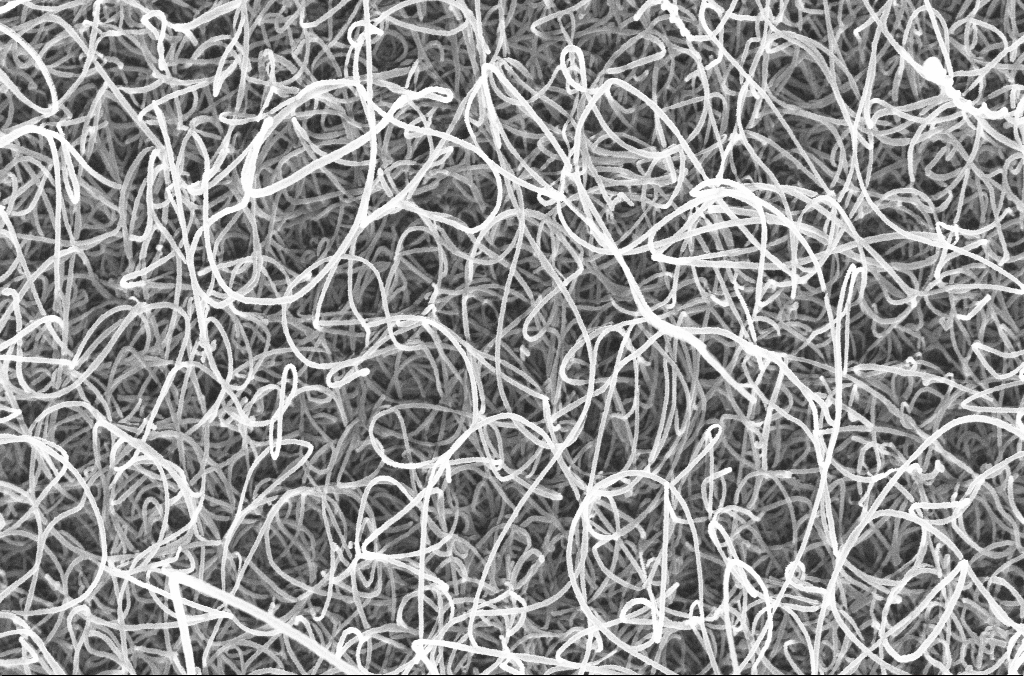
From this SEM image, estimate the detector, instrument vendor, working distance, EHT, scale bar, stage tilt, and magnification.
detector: InLens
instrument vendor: Zeiss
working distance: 4.5 mm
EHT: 20 kV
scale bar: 1000 nm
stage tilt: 0°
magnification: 50 K X